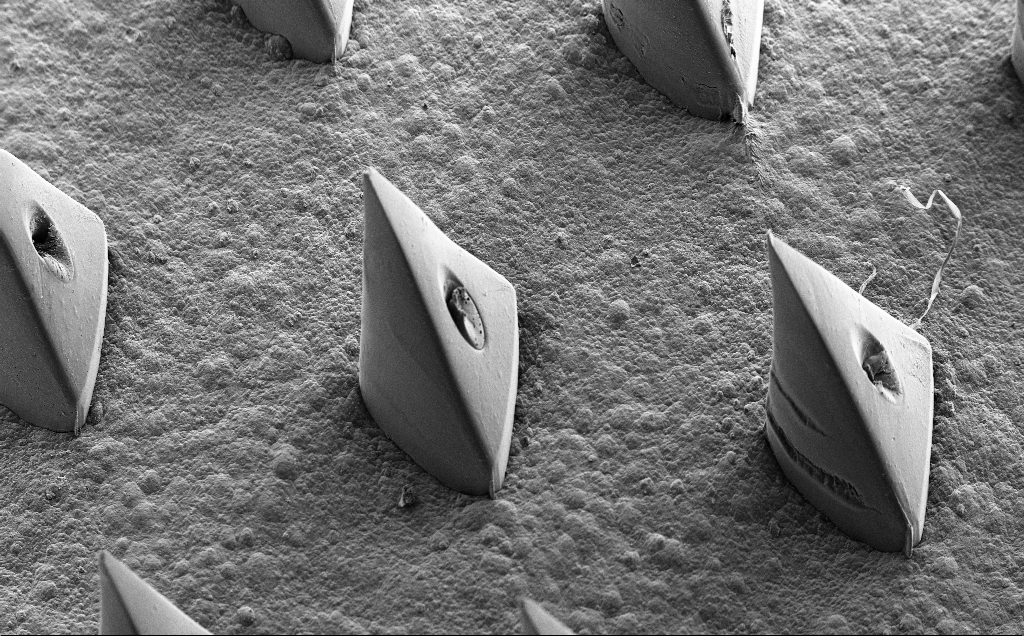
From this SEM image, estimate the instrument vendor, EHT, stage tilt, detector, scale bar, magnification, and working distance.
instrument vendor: Zeiss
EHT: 10 kV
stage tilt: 35.3°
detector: SE2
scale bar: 200000 nm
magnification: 0.1 K X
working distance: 11 mm